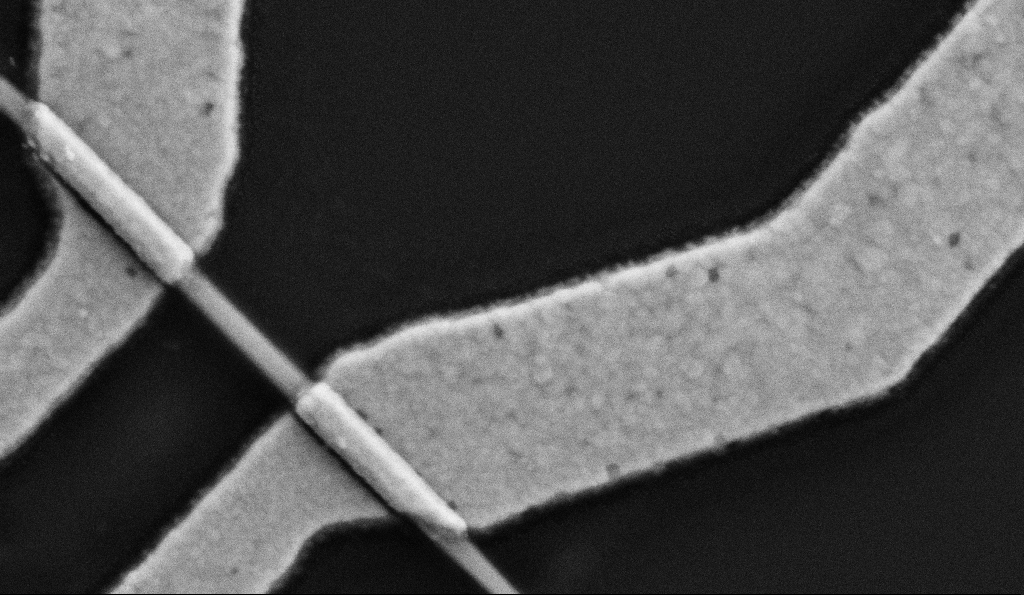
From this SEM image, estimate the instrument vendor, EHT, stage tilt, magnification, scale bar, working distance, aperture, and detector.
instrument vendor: Zeiss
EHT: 5 kV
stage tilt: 0°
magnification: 100 K X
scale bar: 200 nm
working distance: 9.6 mm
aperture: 30 µm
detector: SE2